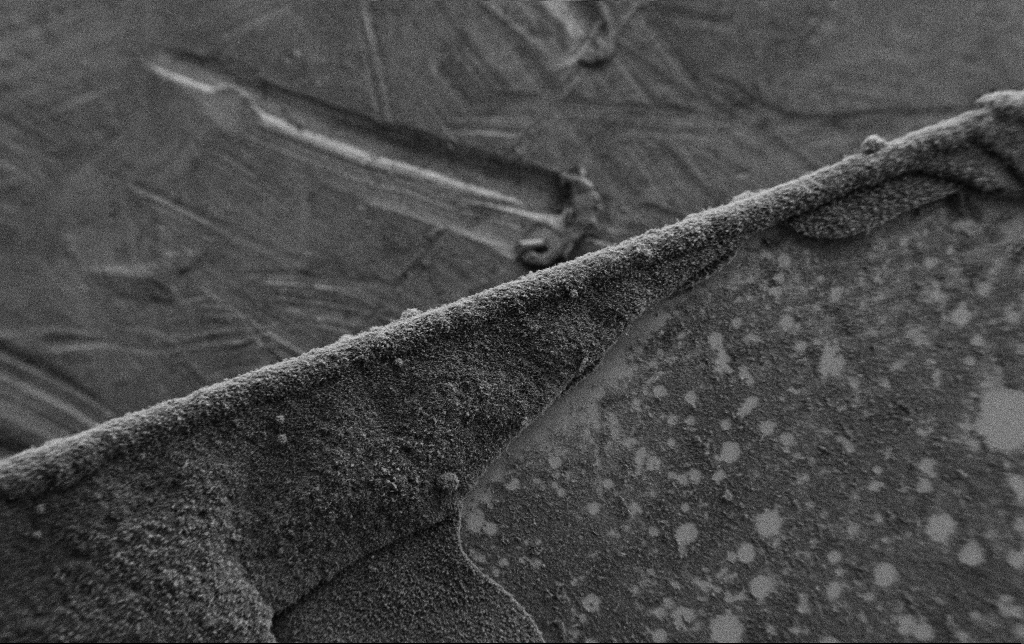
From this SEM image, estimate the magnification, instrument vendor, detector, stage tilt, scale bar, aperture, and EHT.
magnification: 0.2 K X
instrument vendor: Zeiss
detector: SE2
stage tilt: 0°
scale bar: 100000 nm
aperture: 30 µm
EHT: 2 kV